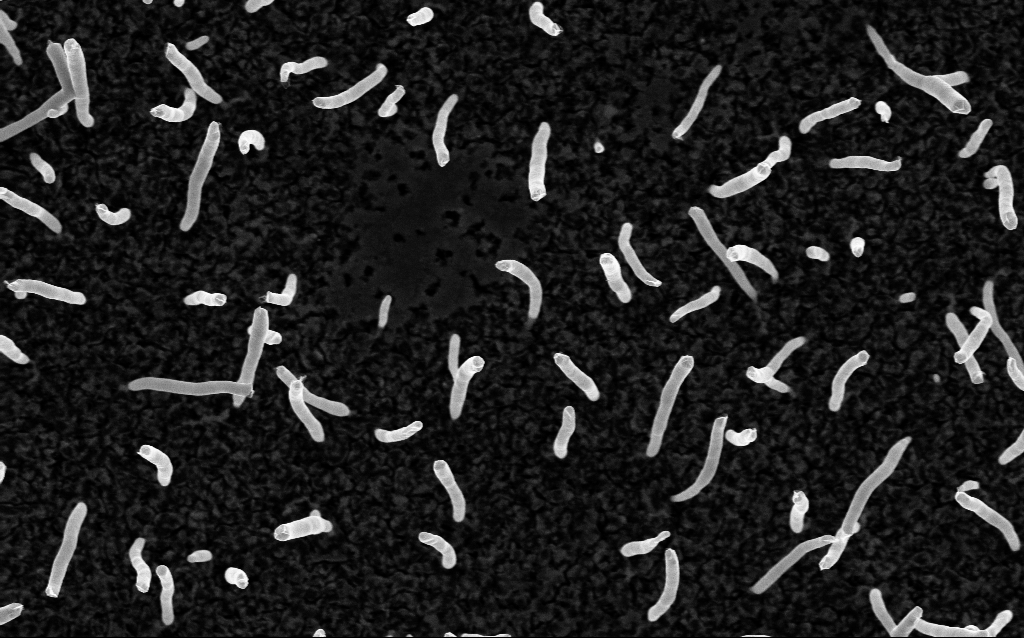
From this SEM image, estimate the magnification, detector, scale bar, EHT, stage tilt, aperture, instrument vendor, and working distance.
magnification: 50 K X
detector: InLens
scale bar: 1000 nm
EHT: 5 kV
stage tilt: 0°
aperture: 30 µm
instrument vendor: Zeiss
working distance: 1.8 mm